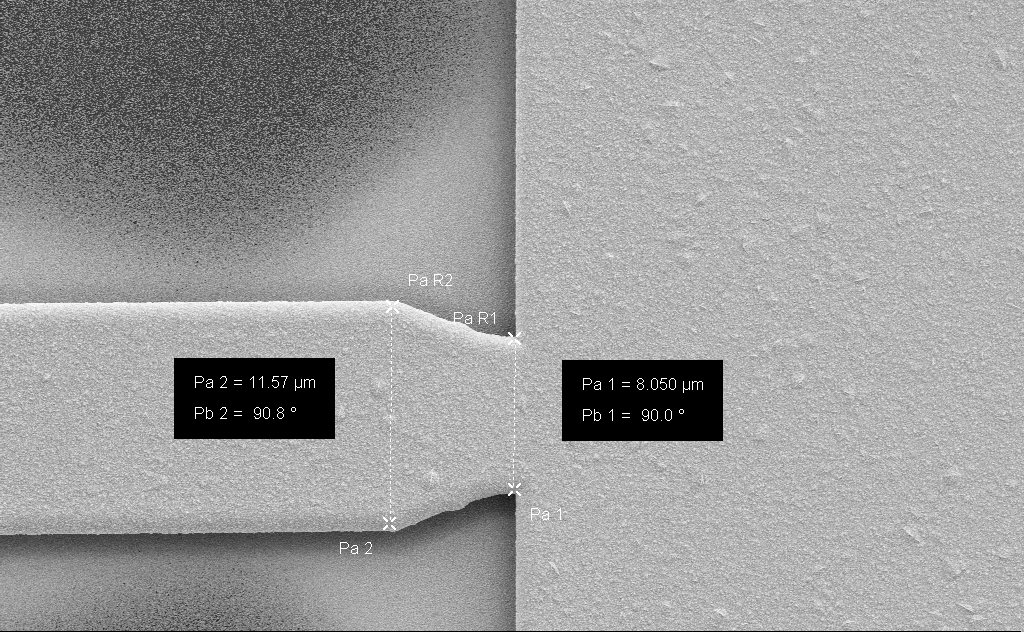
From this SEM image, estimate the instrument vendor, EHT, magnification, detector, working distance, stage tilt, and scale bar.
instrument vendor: Zeiss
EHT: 5 kV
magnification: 6.89 K X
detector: SE2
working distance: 13 mm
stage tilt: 0°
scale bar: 10000 nm